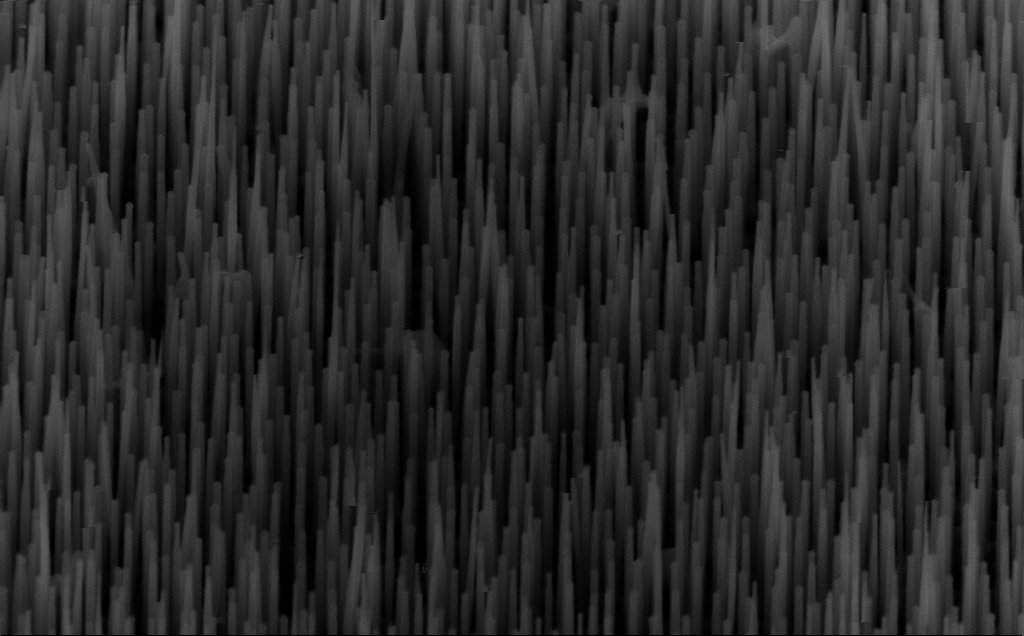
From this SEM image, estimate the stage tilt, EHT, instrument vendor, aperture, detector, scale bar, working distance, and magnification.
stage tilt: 45°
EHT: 10 kV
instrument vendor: Zeiss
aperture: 30 µm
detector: InLens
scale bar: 200 nm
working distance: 5 mm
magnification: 80 K X